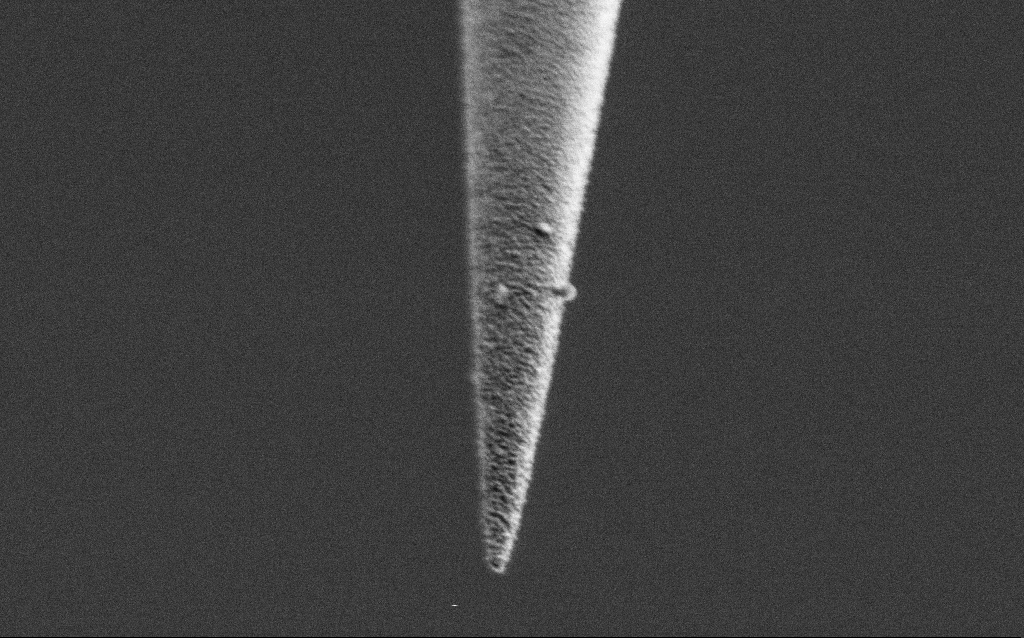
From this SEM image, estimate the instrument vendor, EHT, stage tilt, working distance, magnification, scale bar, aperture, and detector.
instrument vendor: Zeiss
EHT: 1 kV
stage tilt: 45°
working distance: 6.7 mm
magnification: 50 K X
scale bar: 1000 nm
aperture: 30 µm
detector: SE2